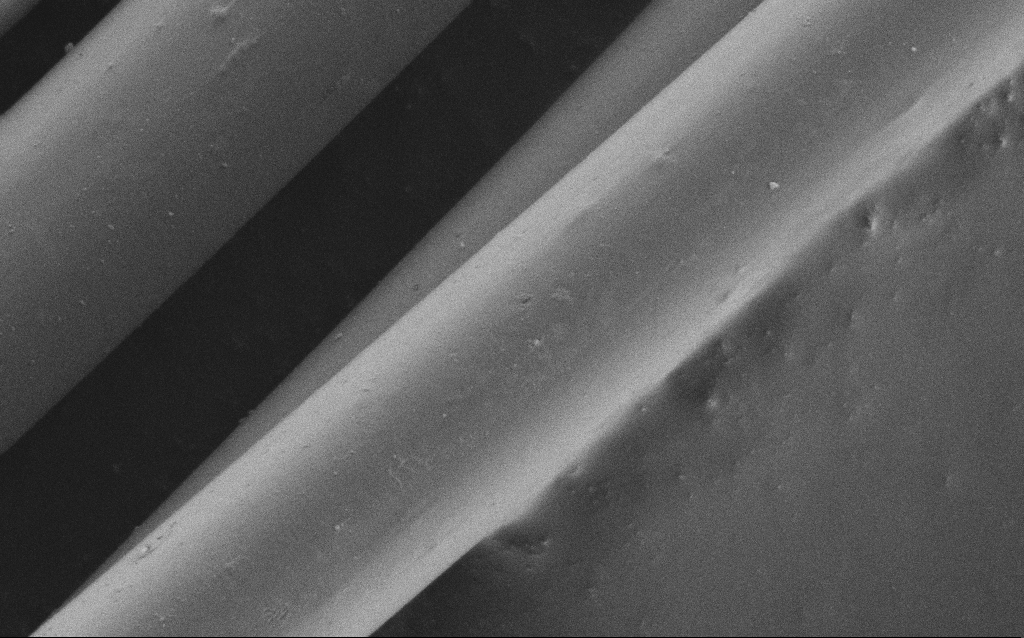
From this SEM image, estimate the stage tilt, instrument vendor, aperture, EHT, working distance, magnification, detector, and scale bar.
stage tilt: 0°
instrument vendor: Zeiss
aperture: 30 µm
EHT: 1 kV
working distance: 5 mm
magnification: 6.41 K X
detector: SE2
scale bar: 10000 nm